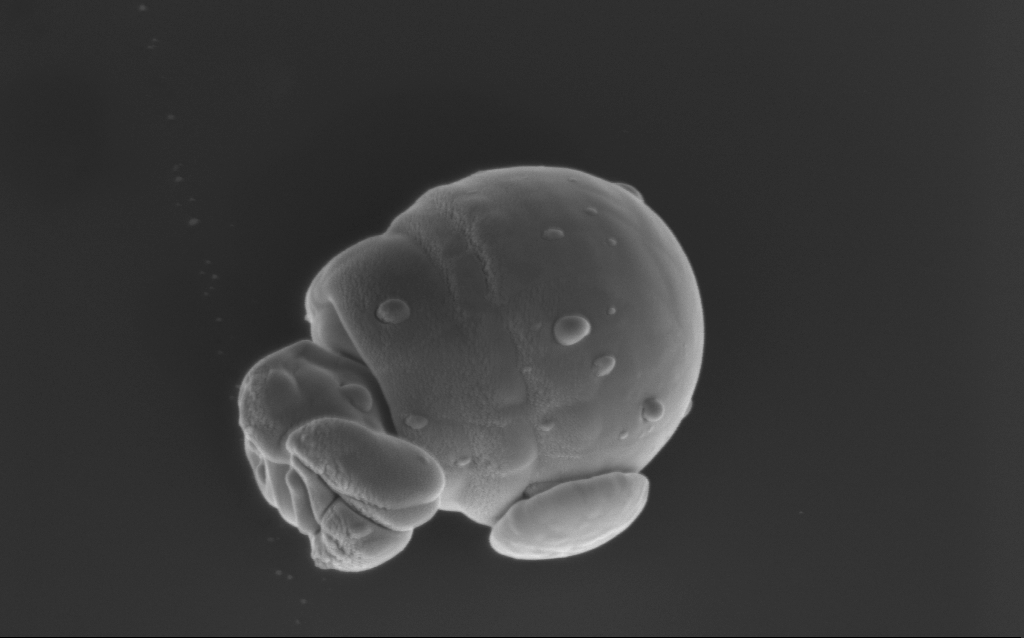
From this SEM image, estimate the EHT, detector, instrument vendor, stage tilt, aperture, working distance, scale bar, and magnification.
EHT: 4 kV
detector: InLens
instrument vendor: Zeiss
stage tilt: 22°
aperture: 30 µm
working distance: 6 mm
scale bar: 1000 nm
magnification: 52.23 K X